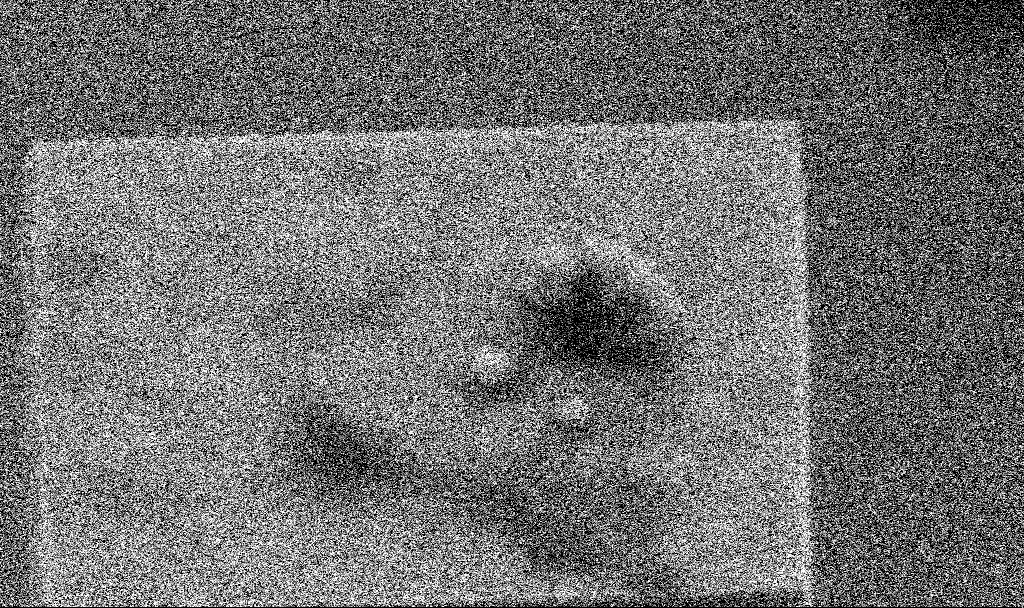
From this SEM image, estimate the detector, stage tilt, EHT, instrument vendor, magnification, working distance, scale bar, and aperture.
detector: SE2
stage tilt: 20°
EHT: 3 kV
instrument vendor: Zeiss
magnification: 62.63 K X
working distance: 5.6 mm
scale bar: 1000 nm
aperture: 30 µm